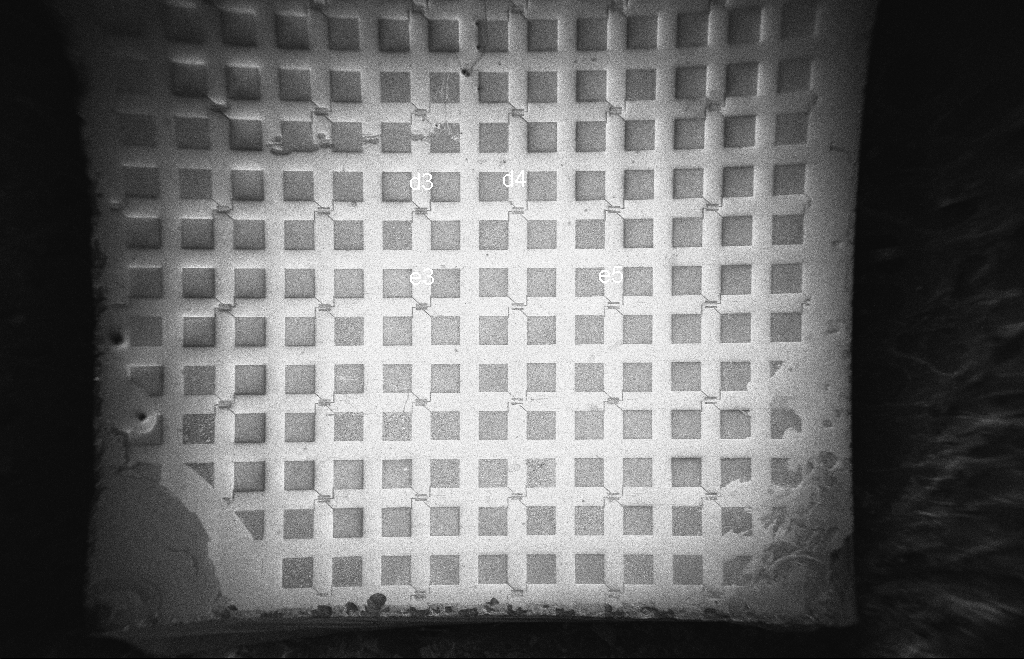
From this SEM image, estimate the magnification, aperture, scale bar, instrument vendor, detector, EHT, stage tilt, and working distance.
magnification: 0.071 K X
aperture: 20 µm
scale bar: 200000 nm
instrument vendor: Zeiss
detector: InLens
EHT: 5 kV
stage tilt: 0°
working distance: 8 mm